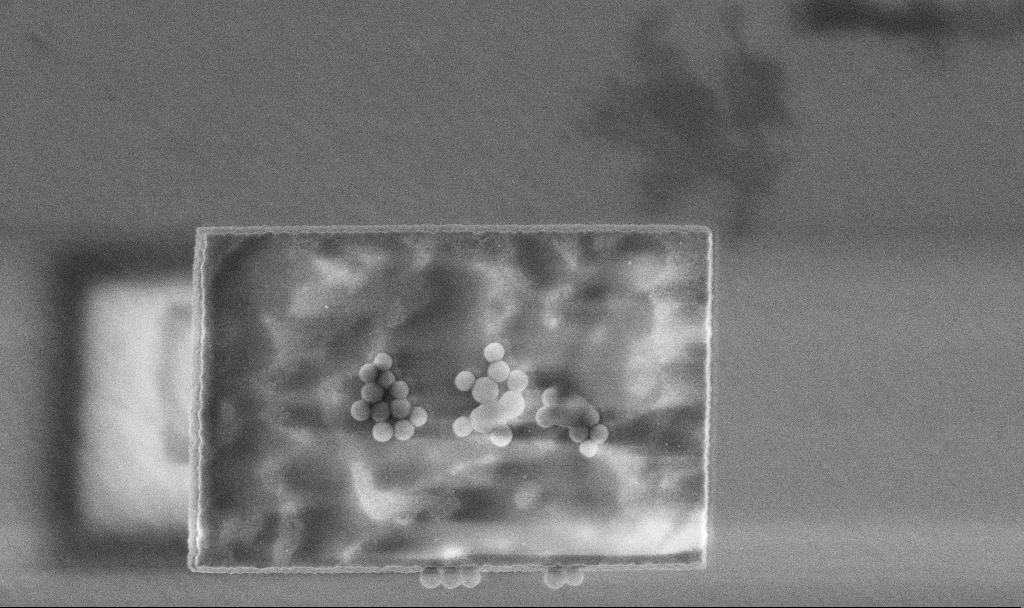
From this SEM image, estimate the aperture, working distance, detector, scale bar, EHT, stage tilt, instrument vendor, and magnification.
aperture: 30 µm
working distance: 3.3 mm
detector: InLens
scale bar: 1000 nm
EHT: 3 kV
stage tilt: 0°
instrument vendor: Zeiss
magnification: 41.87 K X